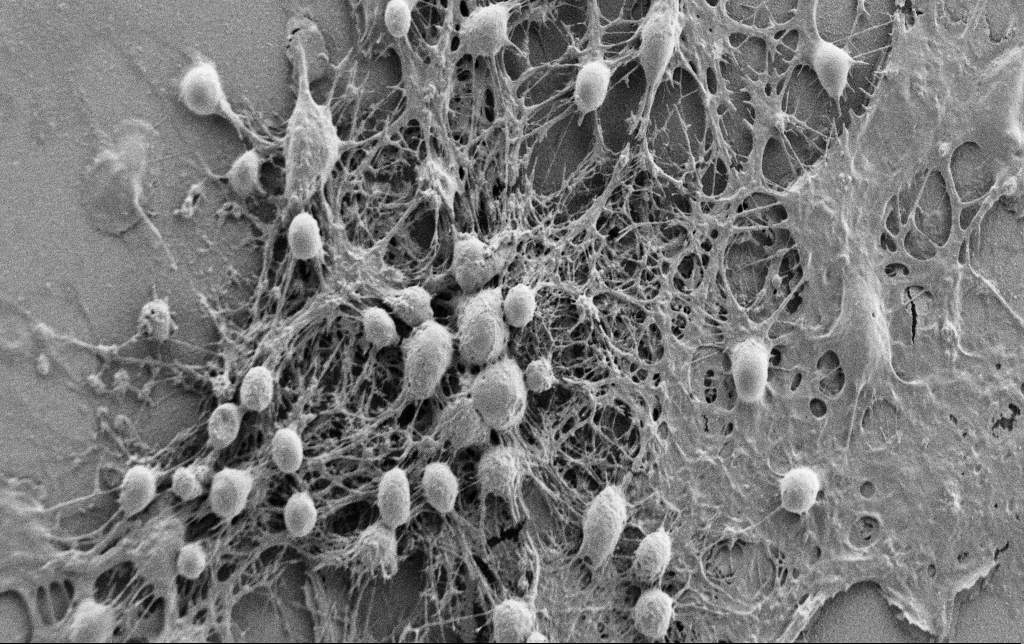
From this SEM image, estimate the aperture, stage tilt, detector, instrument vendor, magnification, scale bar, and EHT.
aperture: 30 µm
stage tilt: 0°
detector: SE2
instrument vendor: Zeiss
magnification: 3 K X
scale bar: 20000 nm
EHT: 0.9 kV